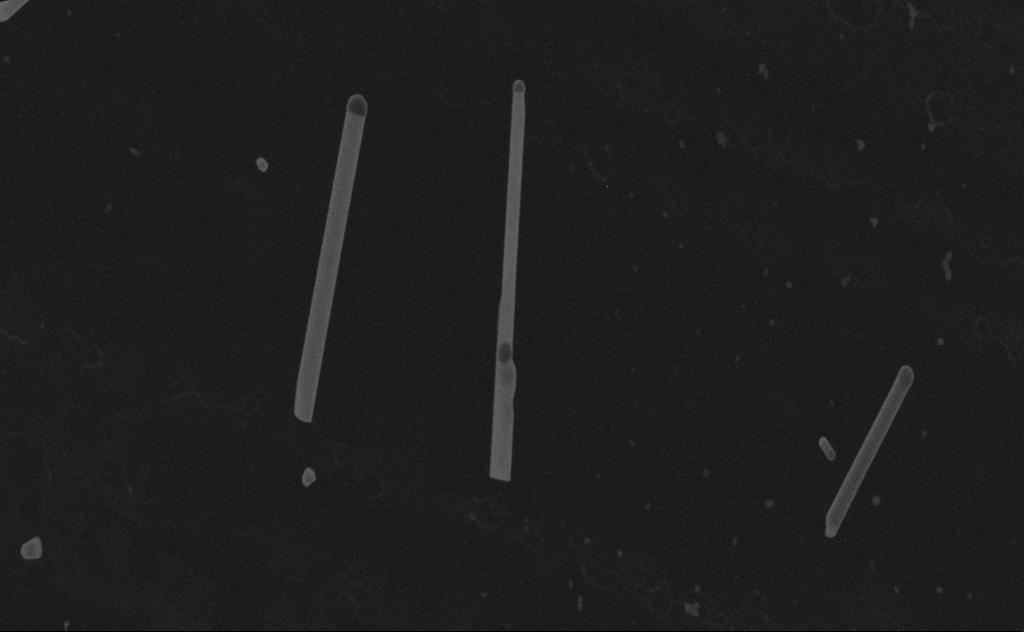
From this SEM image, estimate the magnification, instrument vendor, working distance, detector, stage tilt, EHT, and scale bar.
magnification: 75.67 K X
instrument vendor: Zeiss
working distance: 8 mm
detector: SE2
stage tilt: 0°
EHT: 20 kV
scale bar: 200 nm